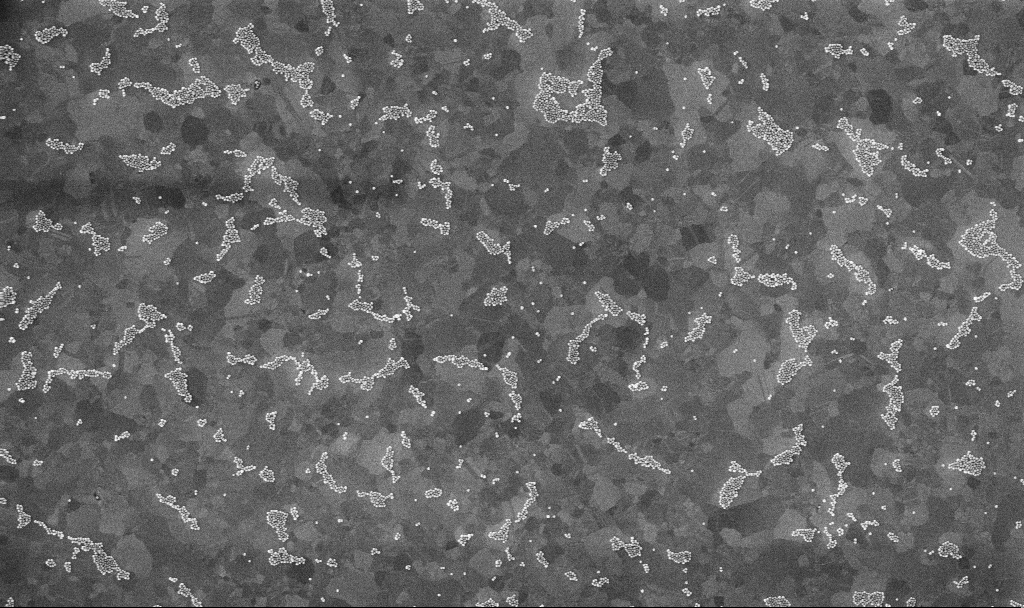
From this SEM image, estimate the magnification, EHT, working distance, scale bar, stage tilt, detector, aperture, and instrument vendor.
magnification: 50 K X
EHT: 10 kV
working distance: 3.4 mm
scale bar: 1000 nm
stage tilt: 0°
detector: InLens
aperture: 30 µm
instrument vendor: Zeiss